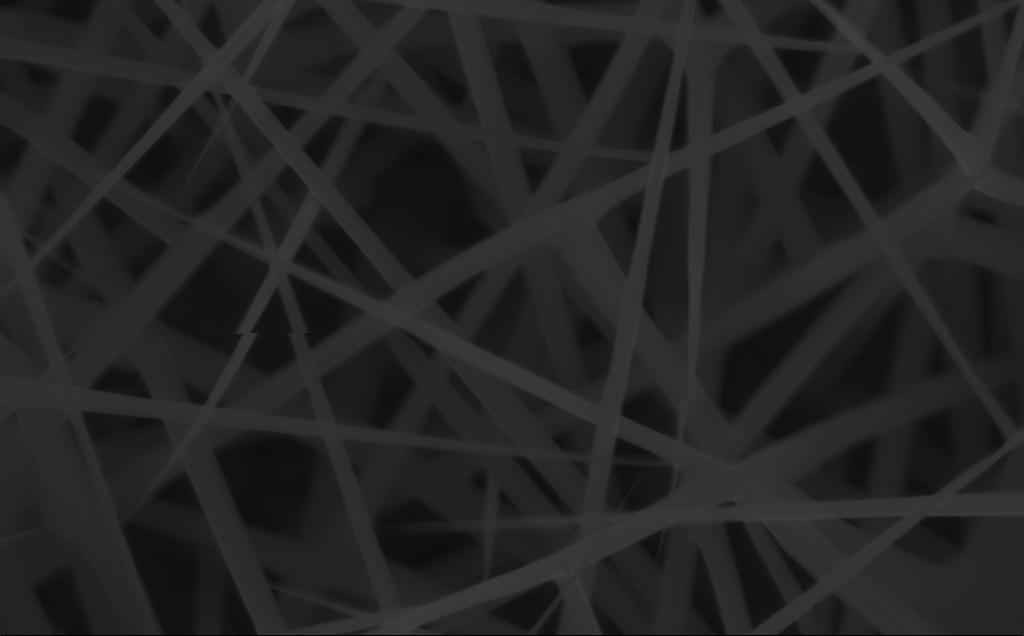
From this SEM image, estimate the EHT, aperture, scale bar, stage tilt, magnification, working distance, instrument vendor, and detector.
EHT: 10 kV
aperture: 30 µm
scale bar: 200 nm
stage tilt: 0°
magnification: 100 K X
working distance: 4 mm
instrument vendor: Zeiss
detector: InLens